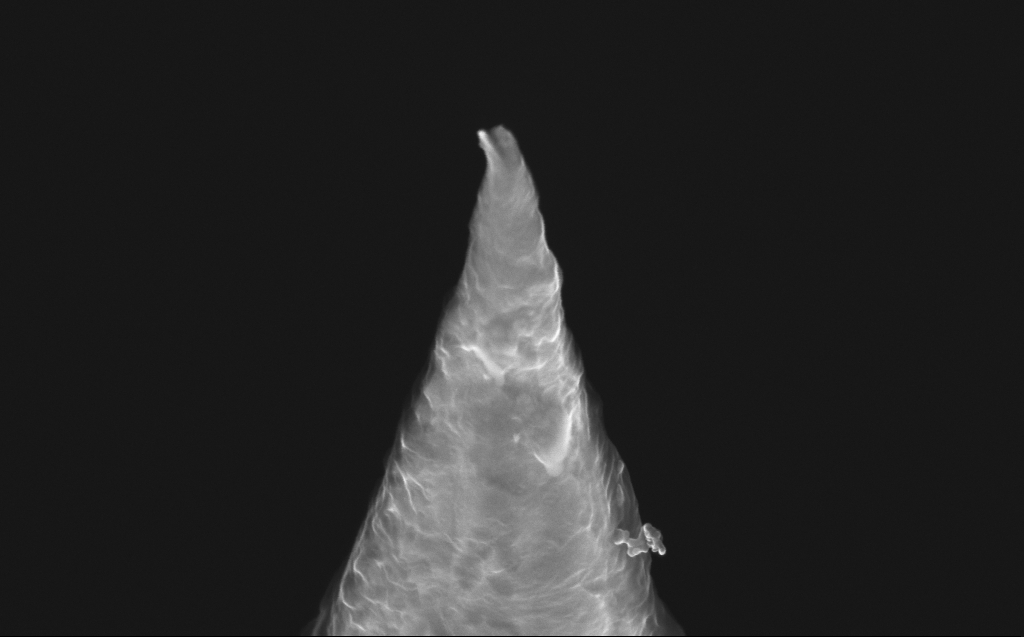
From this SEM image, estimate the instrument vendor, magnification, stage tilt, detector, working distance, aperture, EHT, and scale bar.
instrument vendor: Zeiss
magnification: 100.37 K X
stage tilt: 40°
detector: InLens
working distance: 4 mm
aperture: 30 µm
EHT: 10 kV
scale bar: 200 nm